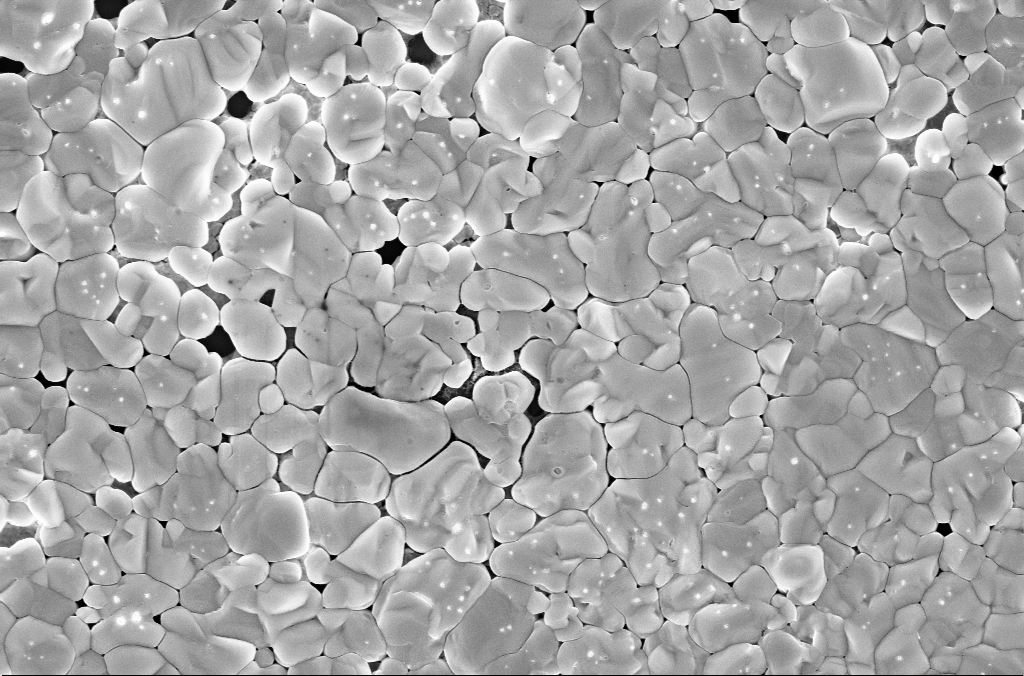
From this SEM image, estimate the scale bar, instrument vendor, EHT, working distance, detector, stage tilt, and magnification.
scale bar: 2000 nm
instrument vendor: Zeiss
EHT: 5 kV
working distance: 3.1 mm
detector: InLens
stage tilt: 0°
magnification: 30 K X